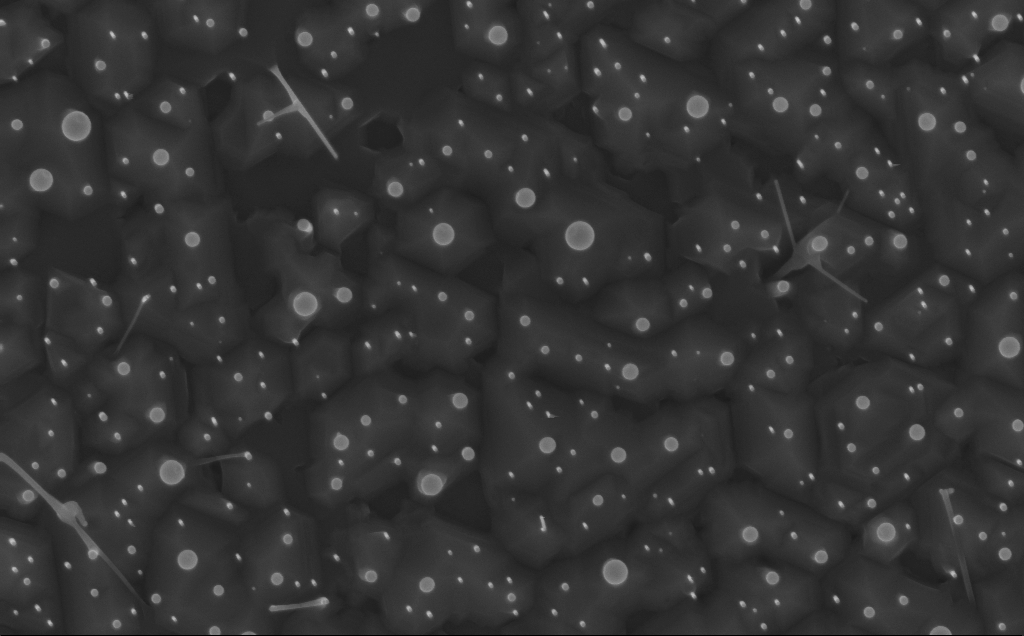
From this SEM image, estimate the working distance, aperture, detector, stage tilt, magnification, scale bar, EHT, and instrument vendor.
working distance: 3 mm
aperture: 30 µm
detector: InLens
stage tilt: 0°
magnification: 40 K X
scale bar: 1000 nm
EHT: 10 kV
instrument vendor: Zeiss